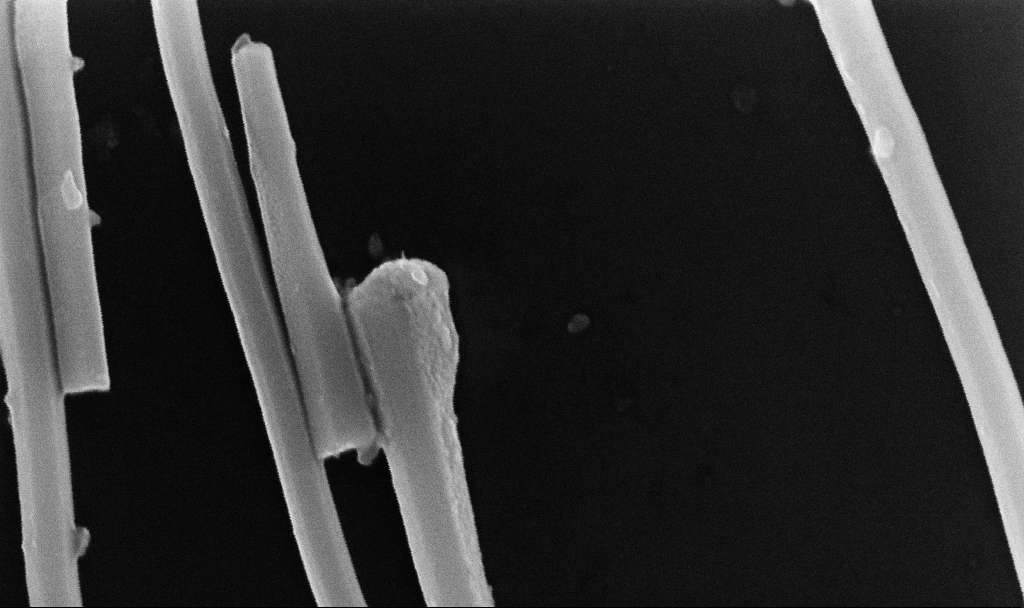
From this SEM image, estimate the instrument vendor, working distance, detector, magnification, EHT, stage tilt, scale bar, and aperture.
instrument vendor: Zeiss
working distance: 8.7 mm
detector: InLens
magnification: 168.08 K X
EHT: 10 kV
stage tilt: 0°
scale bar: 200 nm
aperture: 30 µm